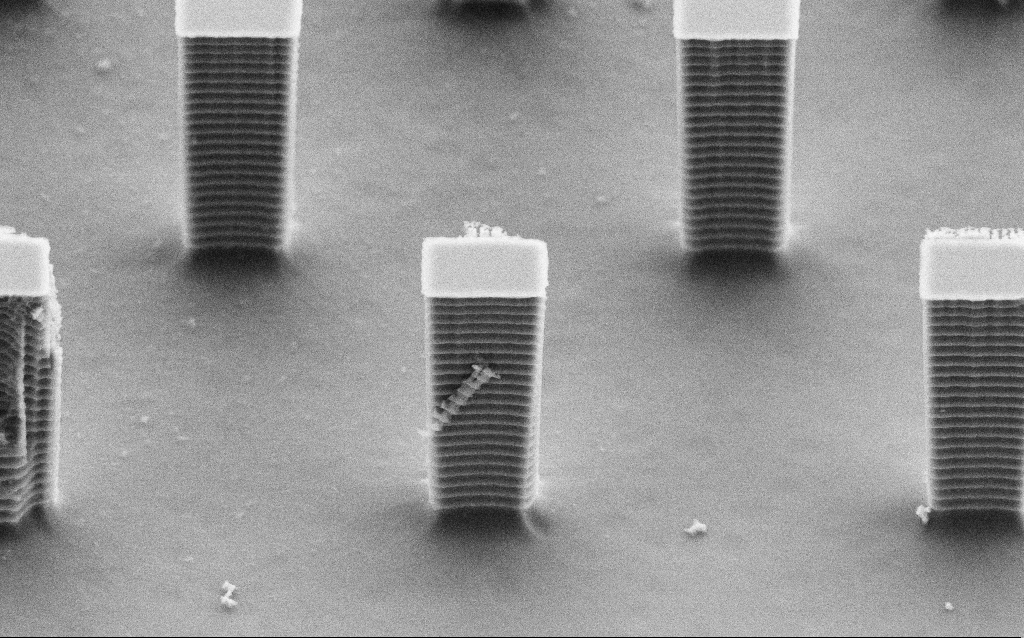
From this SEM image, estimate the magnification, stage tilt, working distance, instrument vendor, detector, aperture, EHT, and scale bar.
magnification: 15.35 K X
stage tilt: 45°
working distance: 6.9 mm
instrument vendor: Zeiss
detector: SE2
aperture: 30 µm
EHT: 5 kV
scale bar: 1000 nm